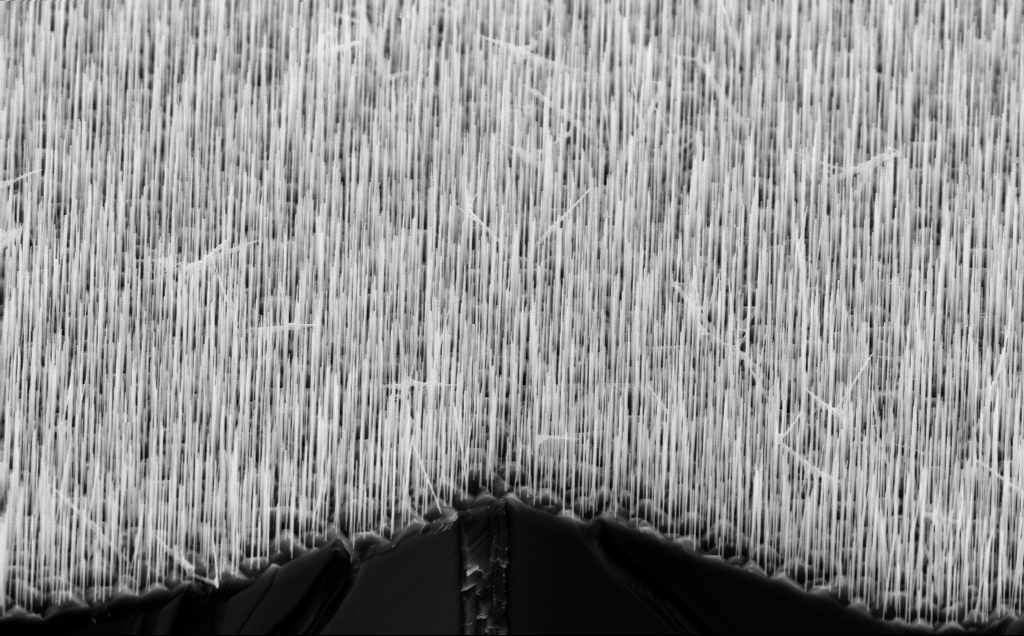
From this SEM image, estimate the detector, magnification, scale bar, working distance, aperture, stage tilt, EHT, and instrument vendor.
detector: InLens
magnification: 13.15 K X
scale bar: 2000 nm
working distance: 5 mm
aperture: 30 µm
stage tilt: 45°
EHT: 10 kV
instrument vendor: Zeiss